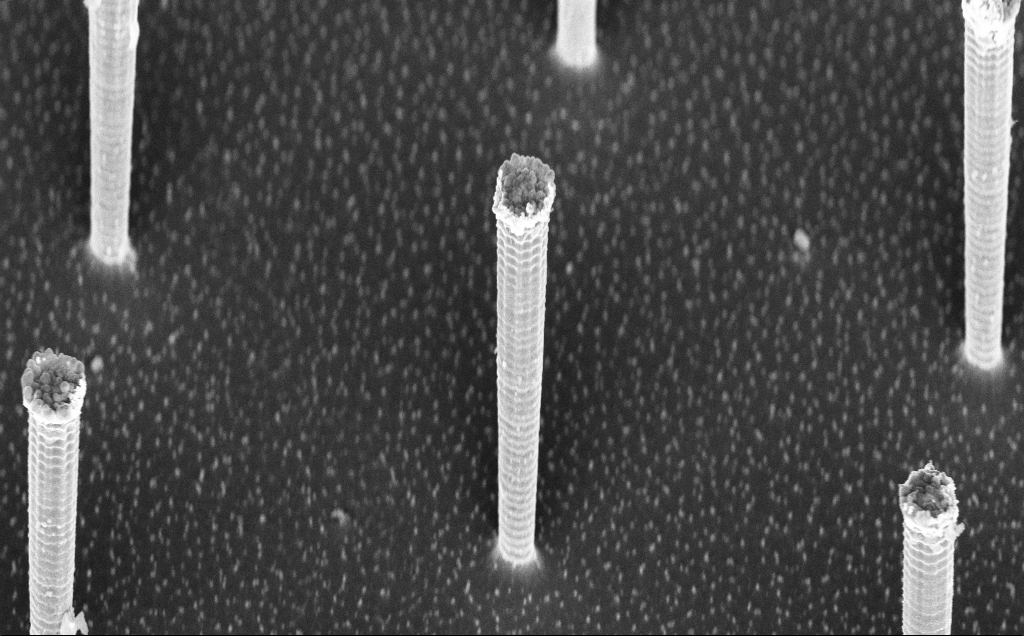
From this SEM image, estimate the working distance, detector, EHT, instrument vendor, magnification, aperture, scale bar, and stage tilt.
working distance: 7 mm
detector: InLens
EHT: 7.5 kV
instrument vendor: Zeiss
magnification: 5.37 K X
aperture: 30 µm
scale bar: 10000 nm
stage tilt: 44.9°